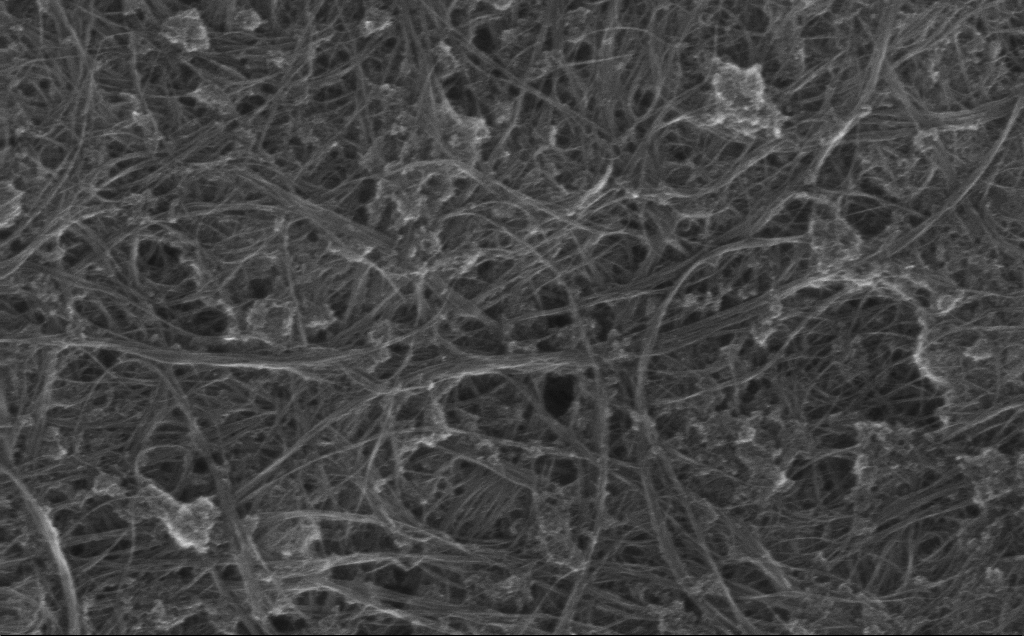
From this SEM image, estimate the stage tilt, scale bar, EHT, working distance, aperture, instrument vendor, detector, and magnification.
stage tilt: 0°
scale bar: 1000 nm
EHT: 10 kV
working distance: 3 mm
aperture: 30 µm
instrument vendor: Zeiss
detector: InLens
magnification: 64.22 K X